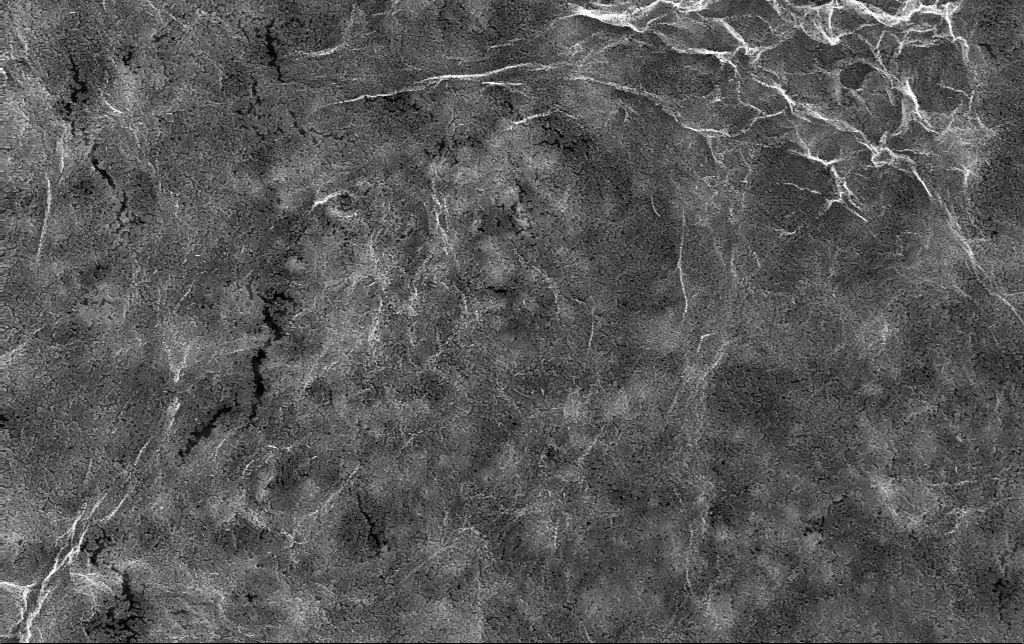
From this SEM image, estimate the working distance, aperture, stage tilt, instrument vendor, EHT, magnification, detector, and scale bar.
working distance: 3 mm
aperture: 30 µm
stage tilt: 0°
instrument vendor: Zeiss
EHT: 10 kV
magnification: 40 K X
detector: InLens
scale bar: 1000 nm